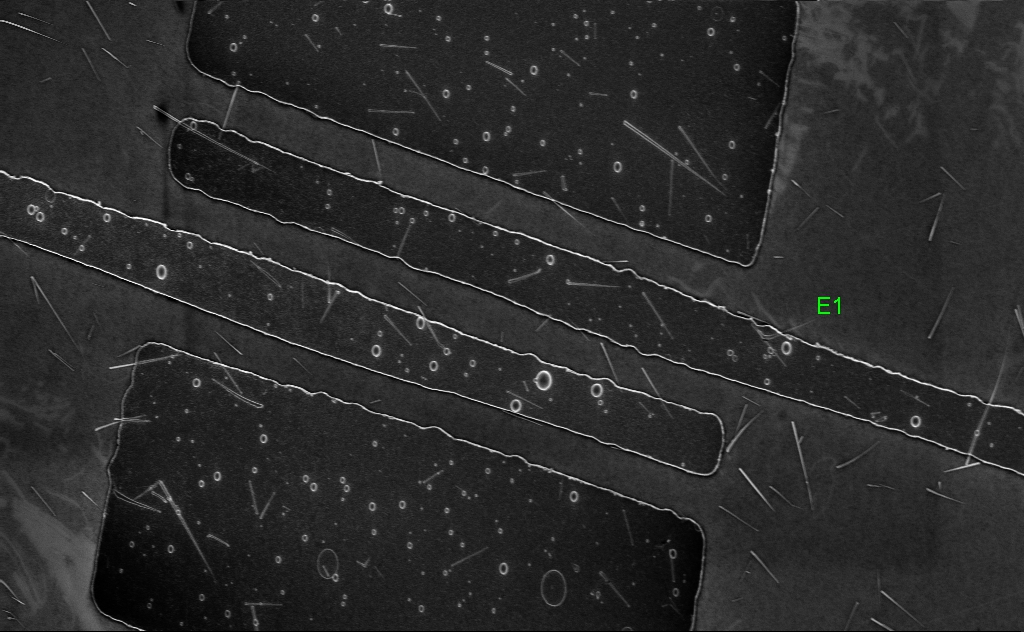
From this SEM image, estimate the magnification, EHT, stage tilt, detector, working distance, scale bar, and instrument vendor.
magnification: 7.51 K X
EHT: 5 kV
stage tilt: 0°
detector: InLens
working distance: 5 mm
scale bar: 2000 nm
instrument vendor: Zeiss